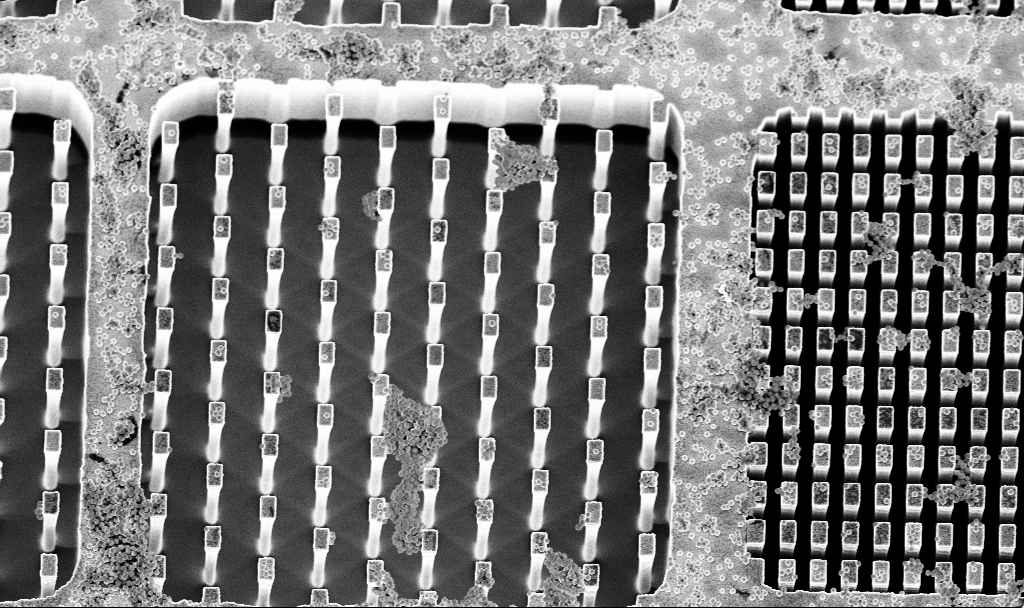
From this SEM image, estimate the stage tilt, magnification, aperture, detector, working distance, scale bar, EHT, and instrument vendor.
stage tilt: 15°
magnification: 3.65 K X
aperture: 30 µm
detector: InLens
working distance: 3.2 mm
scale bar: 10000 nm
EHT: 5 kV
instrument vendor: Zeiss